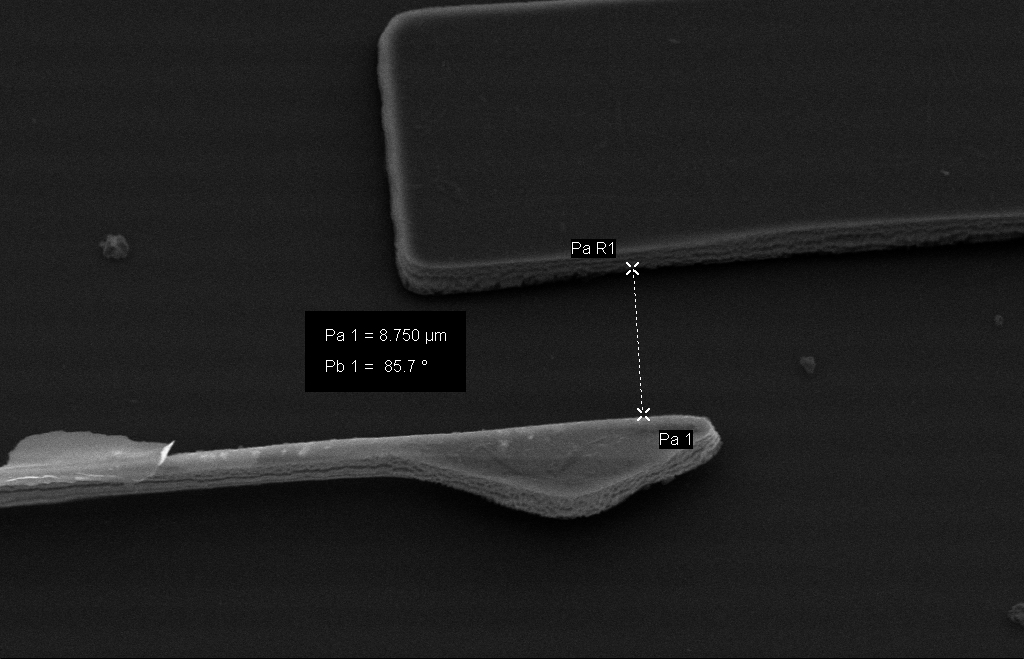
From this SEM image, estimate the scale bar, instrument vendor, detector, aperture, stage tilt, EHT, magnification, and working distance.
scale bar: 2000 nm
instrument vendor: Zeiss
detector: SE2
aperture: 30 µm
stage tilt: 23.3°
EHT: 10 kV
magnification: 6.14 K X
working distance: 20 mm